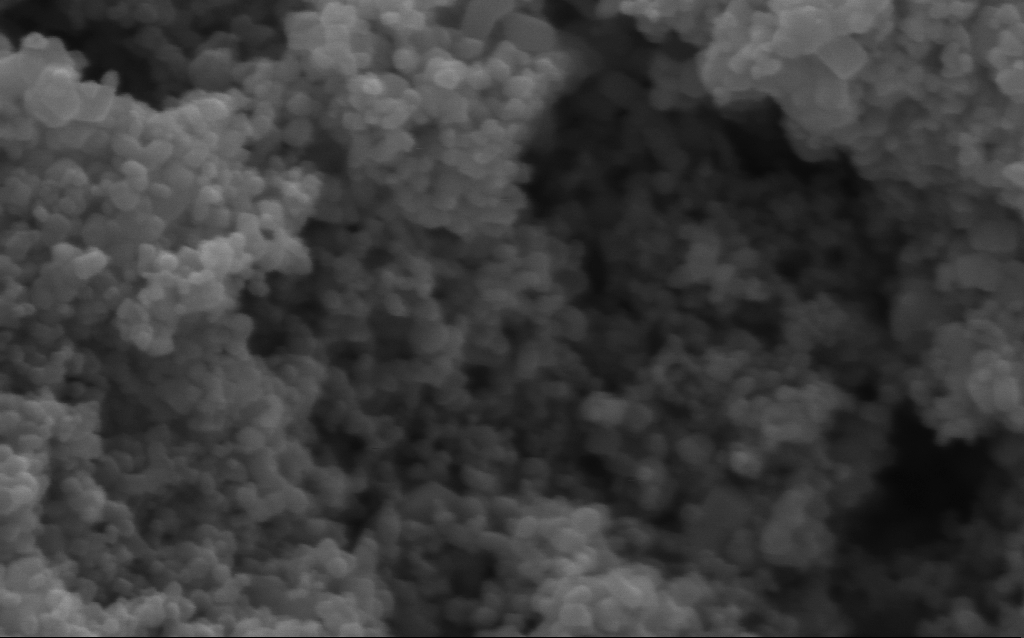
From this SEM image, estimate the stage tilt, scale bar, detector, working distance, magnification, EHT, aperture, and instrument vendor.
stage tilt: -0°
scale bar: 100 nm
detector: InLens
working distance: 4.2 mm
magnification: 294.04 K X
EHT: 5 kV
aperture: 30 µm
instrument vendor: Zeiss